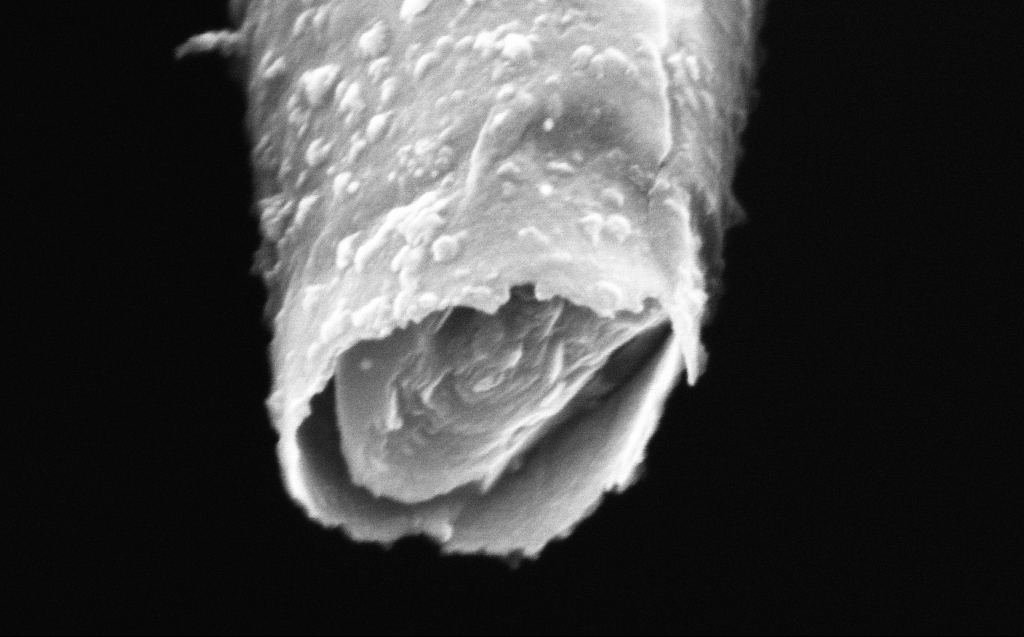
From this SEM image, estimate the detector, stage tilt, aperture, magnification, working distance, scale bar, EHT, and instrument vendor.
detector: InLens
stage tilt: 45°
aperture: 30 µm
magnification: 250 K X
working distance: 4 mm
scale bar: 200 nm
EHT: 4 kV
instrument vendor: Zeiss